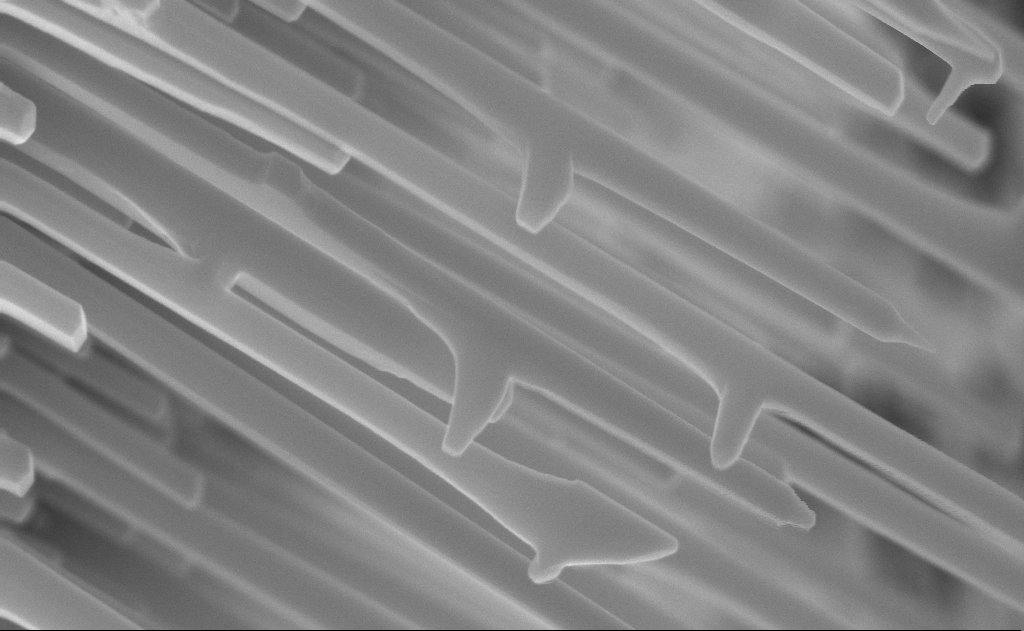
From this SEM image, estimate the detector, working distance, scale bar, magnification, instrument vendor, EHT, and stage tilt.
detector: InLens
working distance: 6 mm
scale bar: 200 nm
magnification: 100 K X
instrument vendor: Zeiss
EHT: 10 kV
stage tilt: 0°